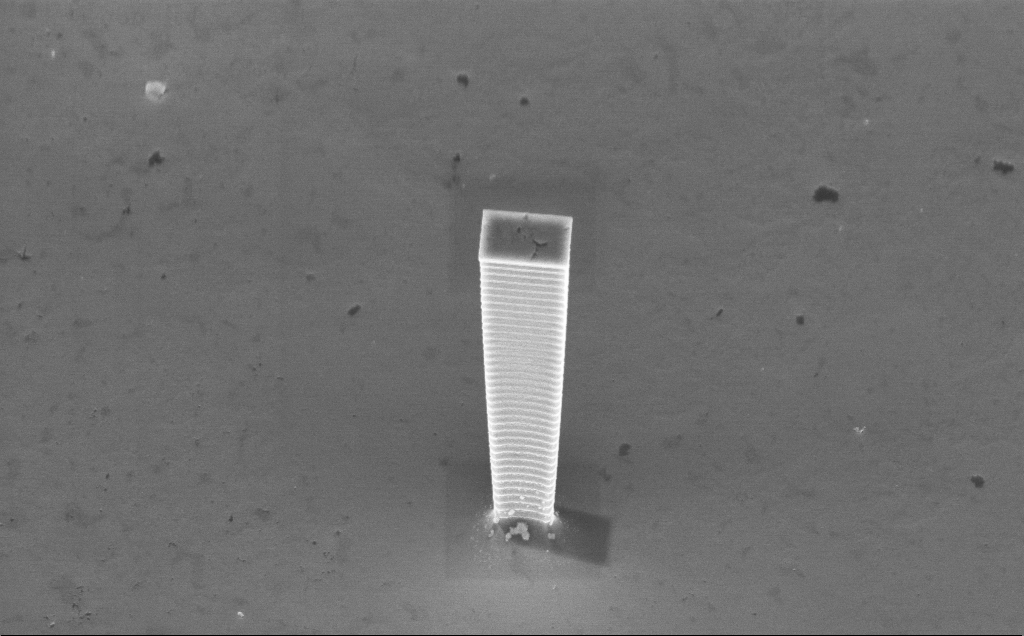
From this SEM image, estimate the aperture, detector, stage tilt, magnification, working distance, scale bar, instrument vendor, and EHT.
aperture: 30 µm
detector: InLens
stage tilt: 45°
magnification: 6.85 K X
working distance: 5 mm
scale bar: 10000 nm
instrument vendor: Zeiss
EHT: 7.5 kV